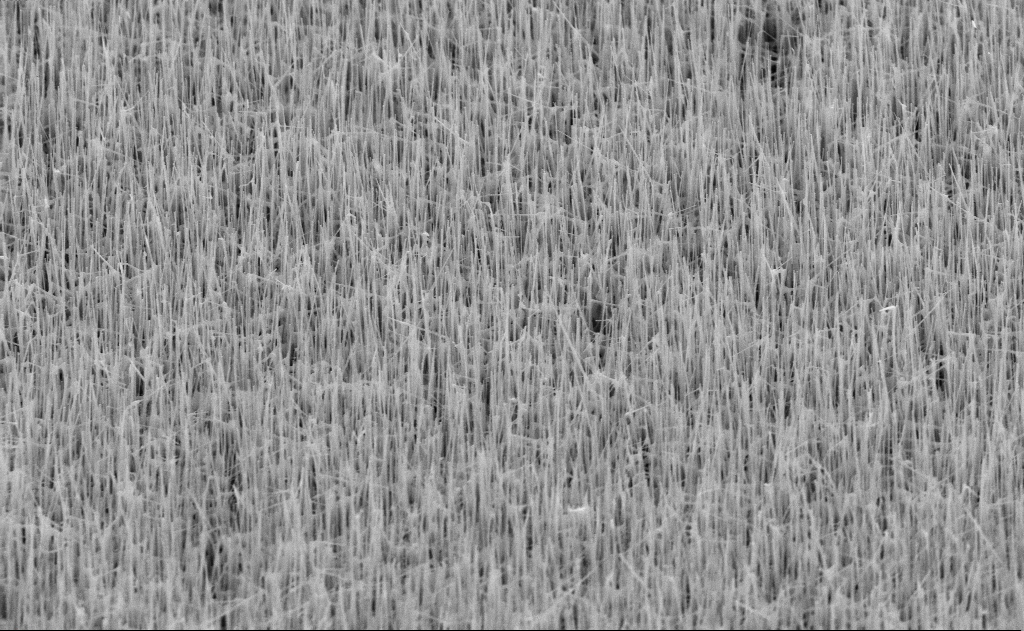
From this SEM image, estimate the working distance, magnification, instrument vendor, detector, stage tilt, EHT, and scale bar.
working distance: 11 mm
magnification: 20 K X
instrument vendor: Zeiss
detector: SE2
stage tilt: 45°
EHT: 10 kV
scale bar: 2000 nm